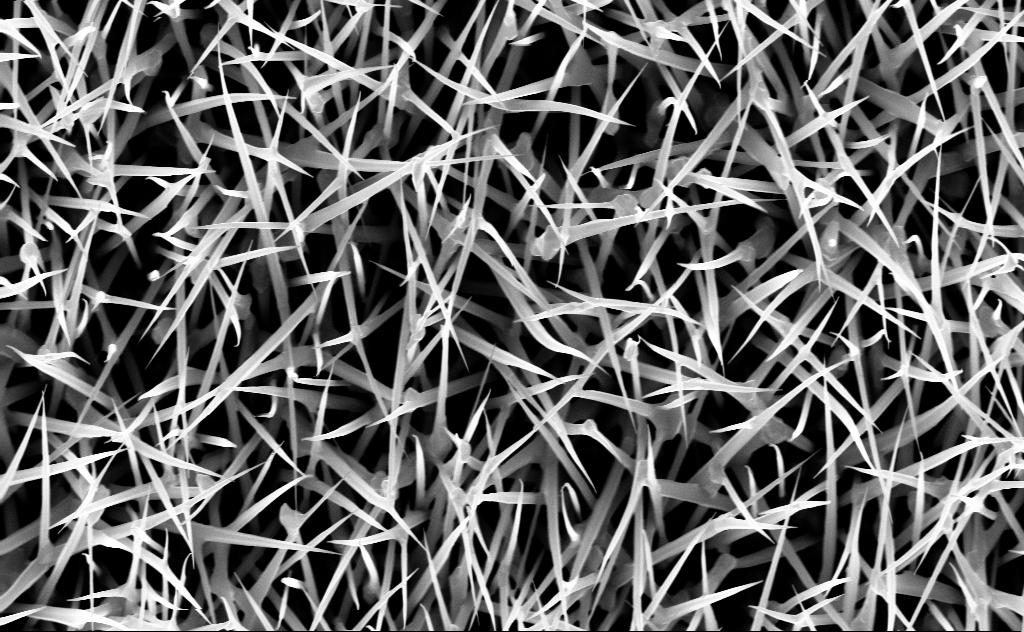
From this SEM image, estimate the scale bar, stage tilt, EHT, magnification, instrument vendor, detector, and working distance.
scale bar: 2000 nm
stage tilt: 0°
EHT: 10 kV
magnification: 20 K X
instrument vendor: Zeiss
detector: InLens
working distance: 6 mm